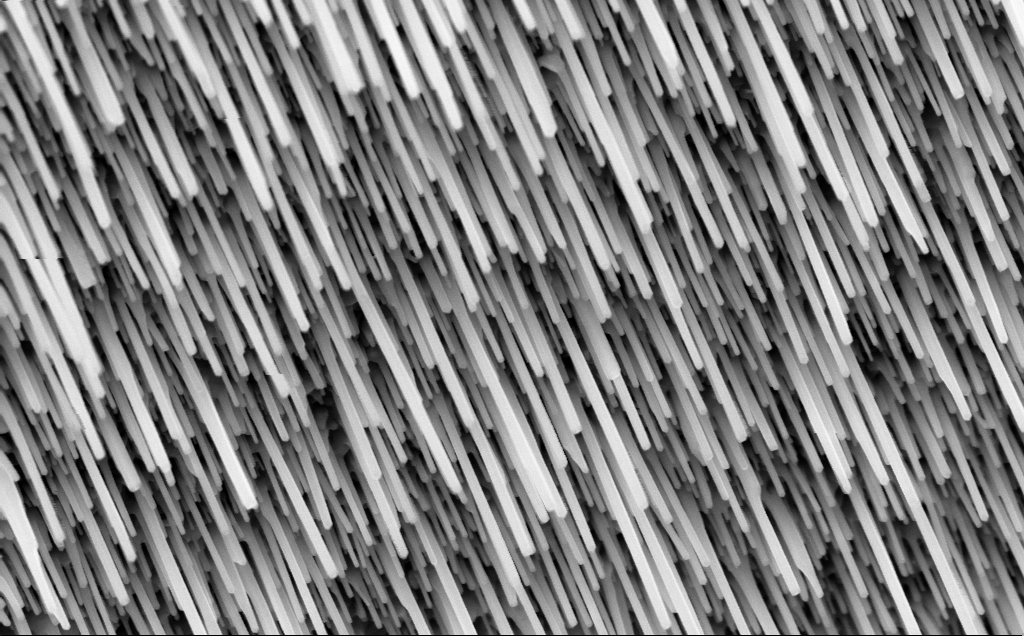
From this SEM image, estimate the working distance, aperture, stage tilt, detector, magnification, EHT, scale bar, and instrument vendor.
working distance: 6 mm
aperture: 30 µm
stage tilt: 0°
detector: InLens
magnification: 73.69 K X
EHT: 10 kV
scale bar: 200 nm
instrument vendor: Zeiss